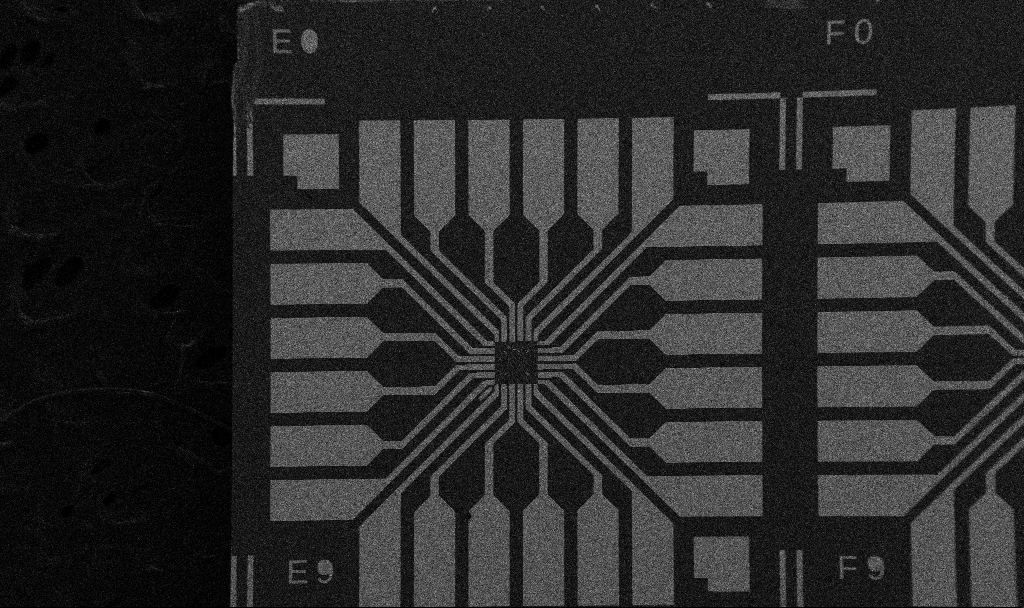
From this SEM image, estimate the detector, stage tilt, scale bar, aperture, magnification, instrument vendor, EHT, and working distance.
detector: SE2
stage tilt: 0°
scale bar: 200000 nm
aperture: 30 µm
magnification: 0.1 K X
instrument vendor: Zeiss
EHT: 5 kV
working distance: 10.7 mm